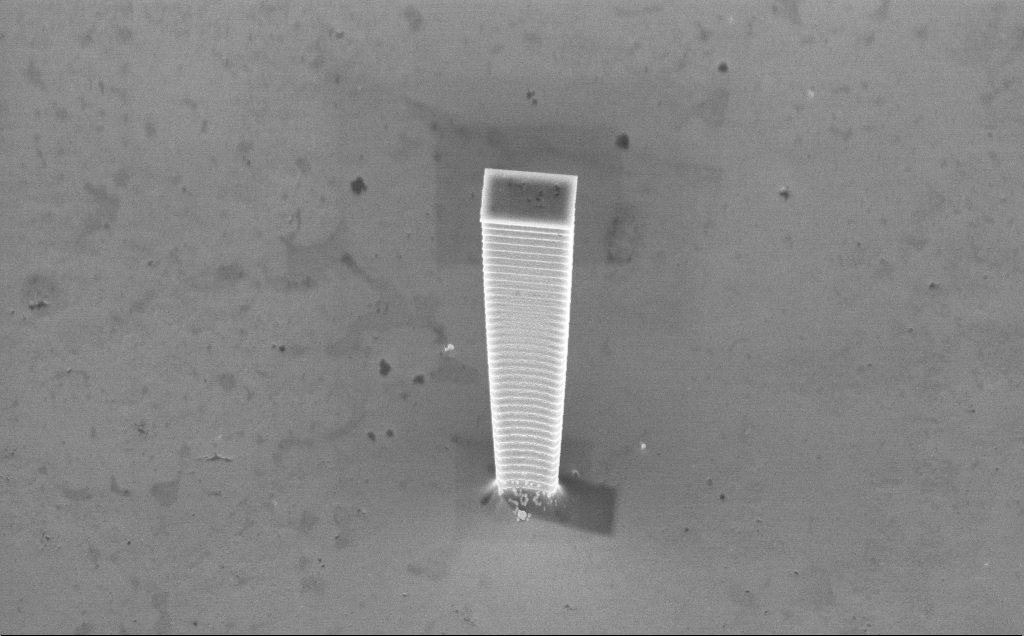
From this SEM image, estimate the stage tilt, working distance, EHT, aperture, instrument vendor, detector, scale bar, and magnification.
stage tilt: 45°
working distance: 5 mm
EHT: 7.5 kV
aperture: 30 µm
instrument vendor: Zeiss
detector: InLens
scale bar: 10000 nm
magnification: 7.09 K X